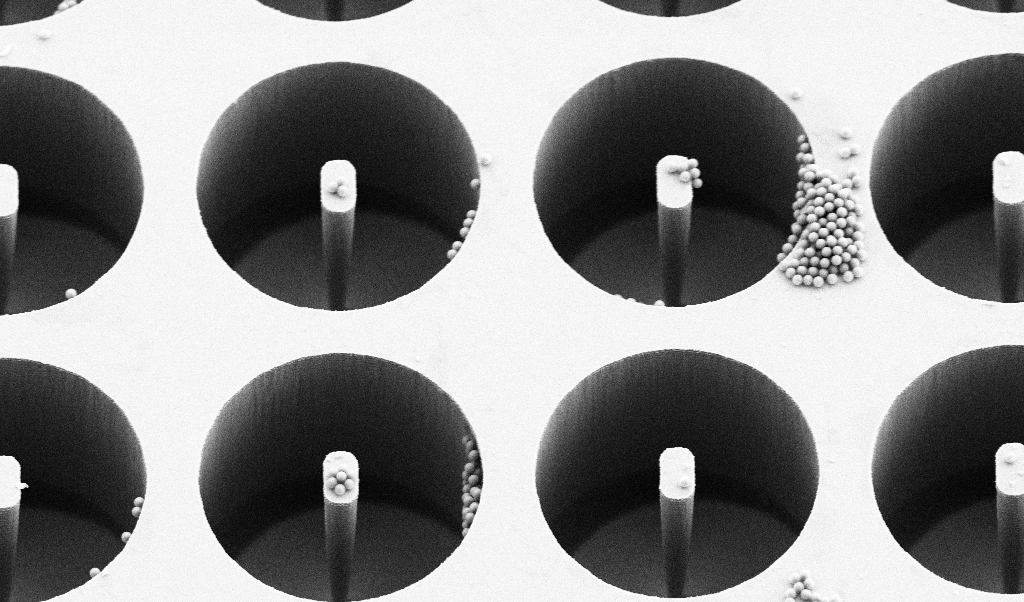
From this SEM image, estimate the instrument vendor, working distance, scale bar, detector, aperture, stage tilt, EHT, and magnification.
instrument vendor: Zeiss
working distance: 7.1 mm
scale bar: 10000 nm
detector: SE2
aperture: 30 µm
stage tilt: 30°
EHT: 5 kV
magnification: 5.36 K X